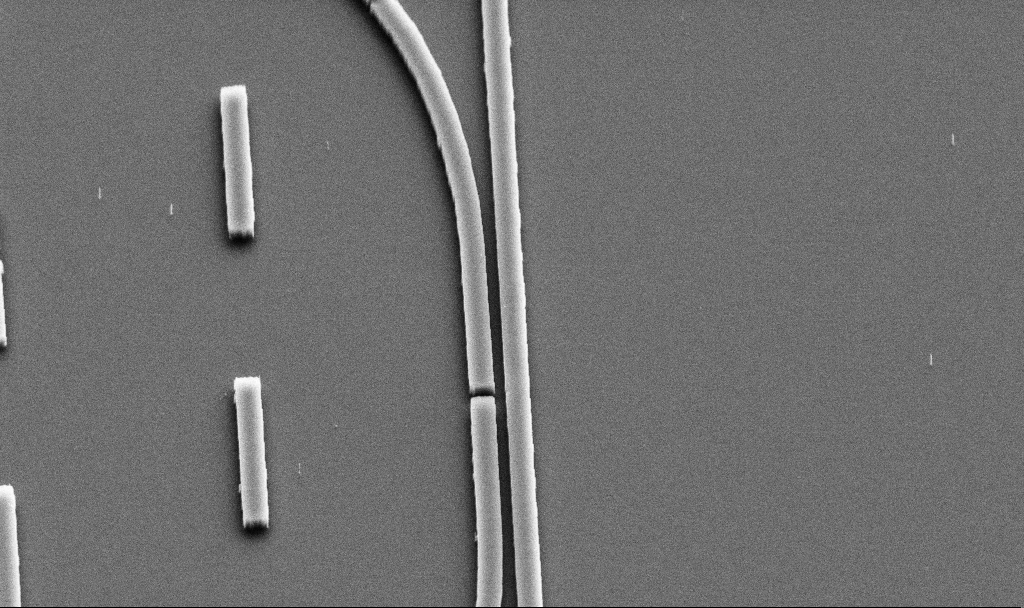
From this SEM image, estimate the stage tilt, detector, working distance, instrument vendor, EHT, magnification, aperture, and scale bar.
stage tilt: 45°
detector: SE2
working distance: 9.8 mm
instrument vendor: Zeiss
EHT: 5 kV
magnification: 18.18 K X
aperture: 30 µm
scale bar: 1000 nm